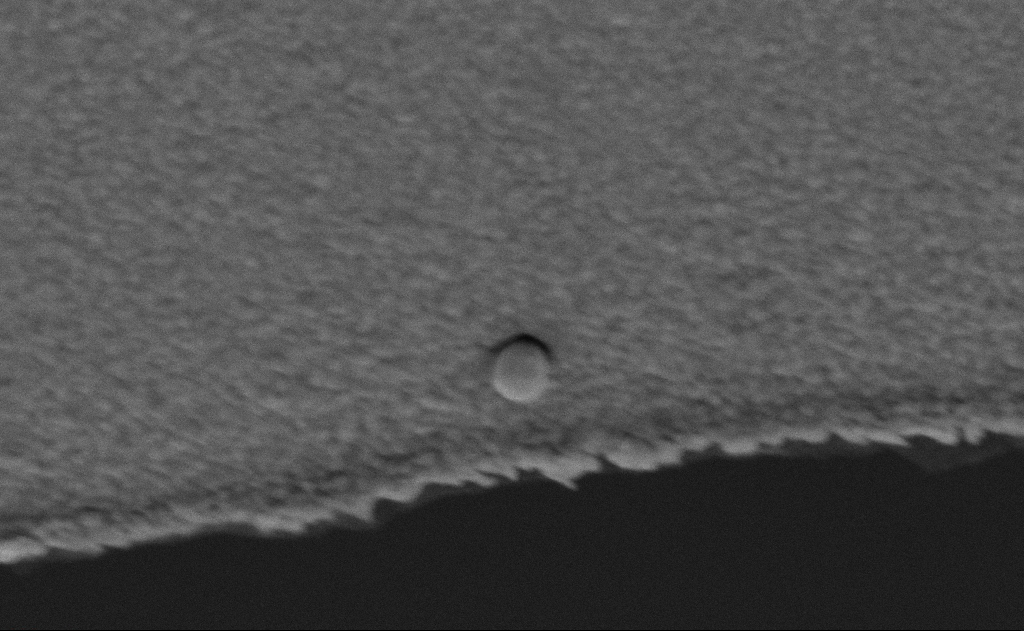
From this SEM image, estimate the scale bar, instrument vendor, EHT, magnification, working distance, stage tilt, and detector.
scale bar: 200 nm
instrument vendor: Zeiss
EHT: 5 kV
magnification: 130.6 K X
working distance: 8 mm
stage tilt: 39.8°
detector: SE2